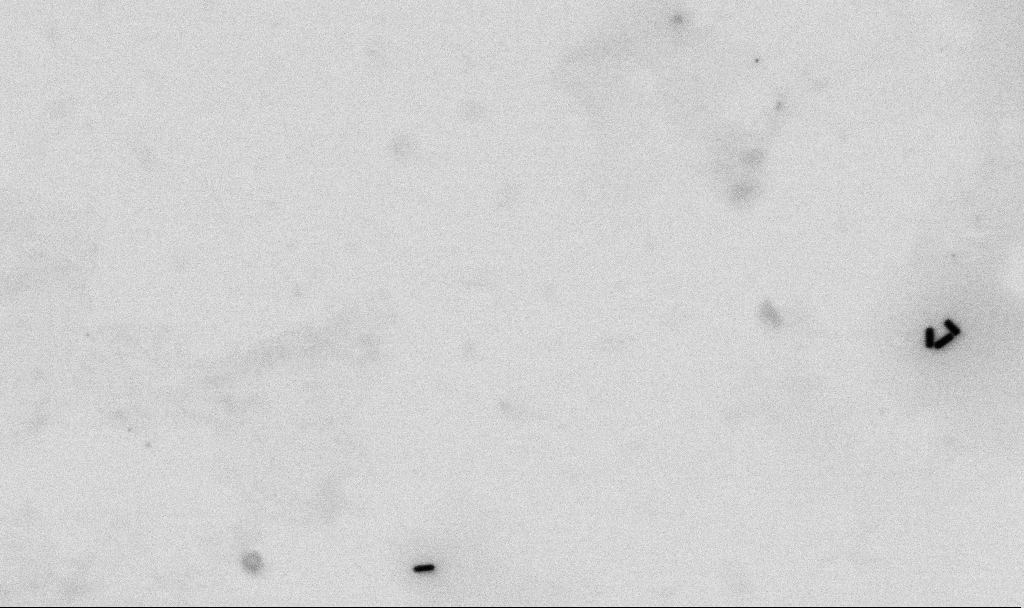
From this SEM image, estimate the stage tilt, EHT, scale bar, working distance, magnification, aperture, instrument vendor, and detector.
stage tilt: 0°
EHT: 2 kV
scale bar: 200 nm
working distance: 4.5 mm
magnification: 100 K X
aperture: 30 µm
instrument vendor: Zeiss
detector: SE2